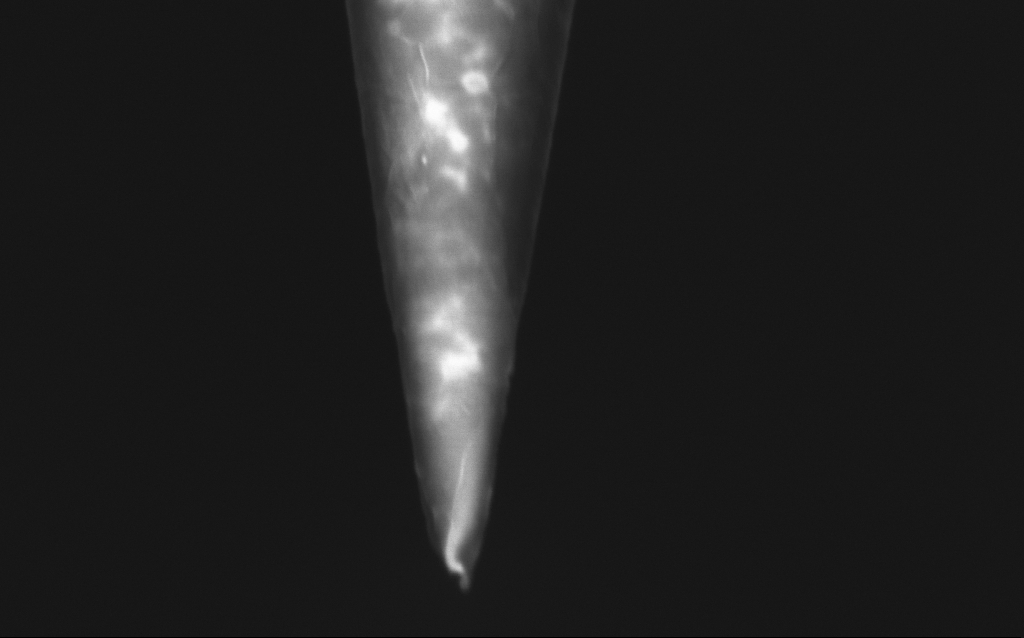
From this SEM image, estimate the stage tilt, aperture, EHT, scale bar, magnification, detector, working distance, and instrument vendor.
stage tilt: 45°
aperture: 30 µm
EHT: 1 kV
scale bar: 200 nm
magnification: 100 K X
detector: InLens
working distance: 6 mm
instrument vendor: Zeiss